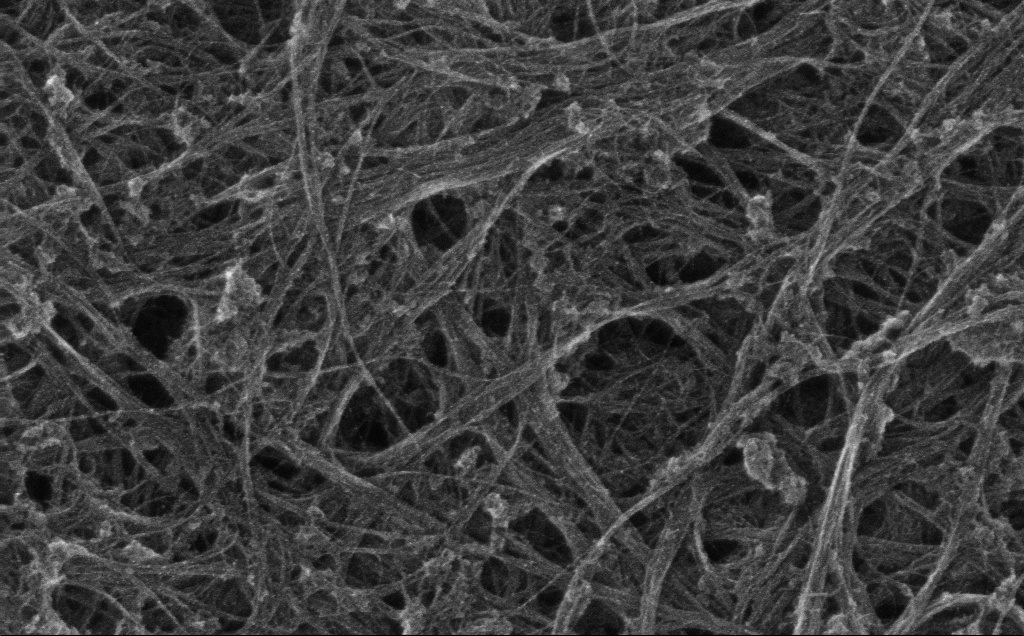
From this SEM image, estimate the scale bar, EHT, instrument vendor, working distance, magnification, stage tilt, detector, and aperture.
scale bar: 200 nm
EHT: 10 kV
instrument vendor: Zeiss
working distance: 3 mm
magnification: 89.01 K X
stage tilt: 0°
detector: InLens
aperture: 30 µm